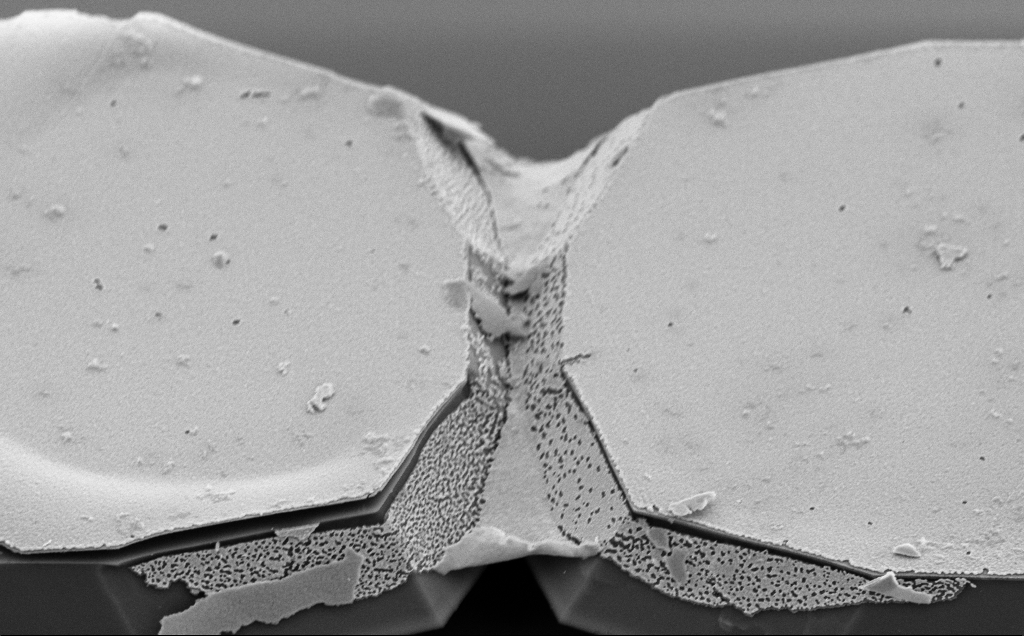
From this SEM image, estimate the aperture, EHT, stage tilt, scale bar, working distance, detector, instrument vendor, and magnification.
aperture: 30 µm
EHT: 5 kV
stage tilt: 50°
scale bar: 2000 nm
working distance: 10 mm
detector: SE2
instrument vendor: Zeiss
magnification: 16.67 K X